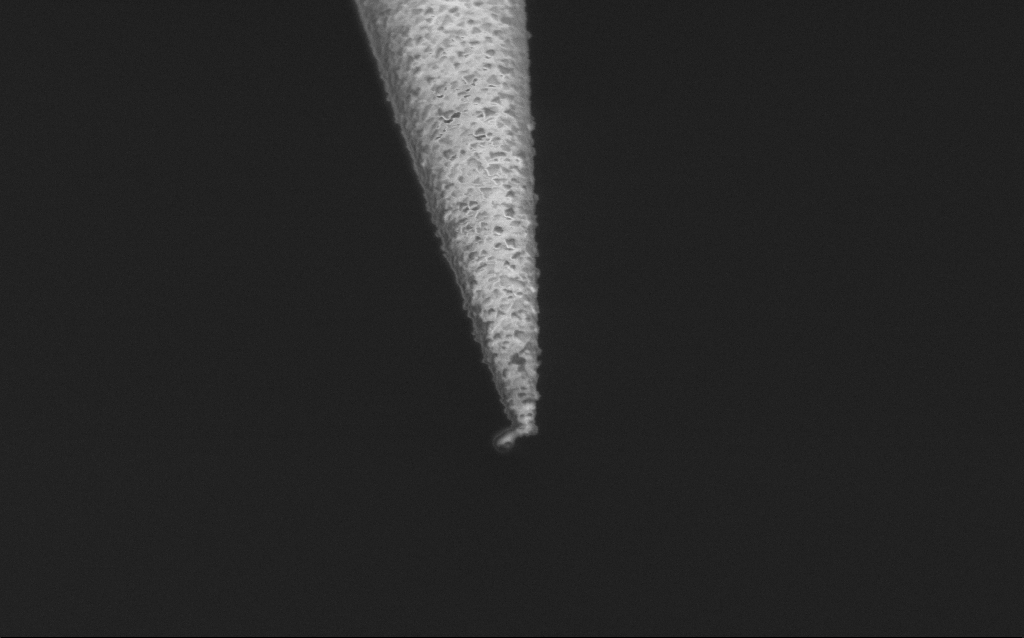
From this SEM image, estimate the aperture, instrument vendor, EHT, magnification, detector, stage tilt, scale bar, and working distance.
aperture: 30 µm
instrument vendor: Zeiss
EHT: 2 kV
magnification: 50 K X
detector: InLens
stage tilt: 45°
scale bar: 1000 nm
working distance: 7.6 mm